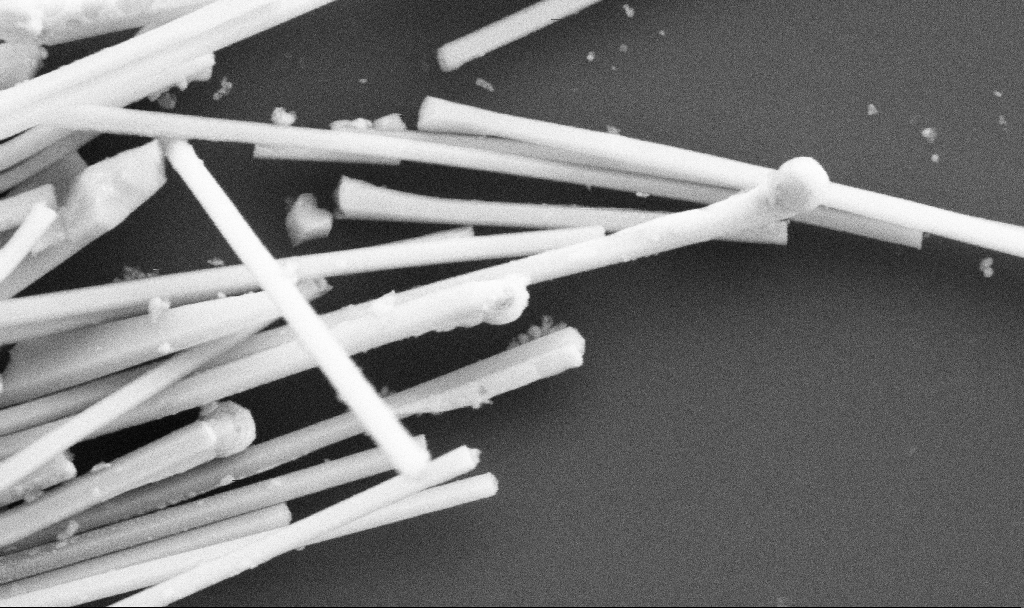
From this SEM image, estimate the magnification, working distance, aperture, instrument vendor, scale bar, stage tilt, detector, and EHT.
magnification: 96.13 K X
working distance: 6.7 mm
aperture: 30 µm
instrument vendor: Zeiss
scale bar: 200 nm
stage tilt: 0°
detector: SE2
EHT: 5 kV